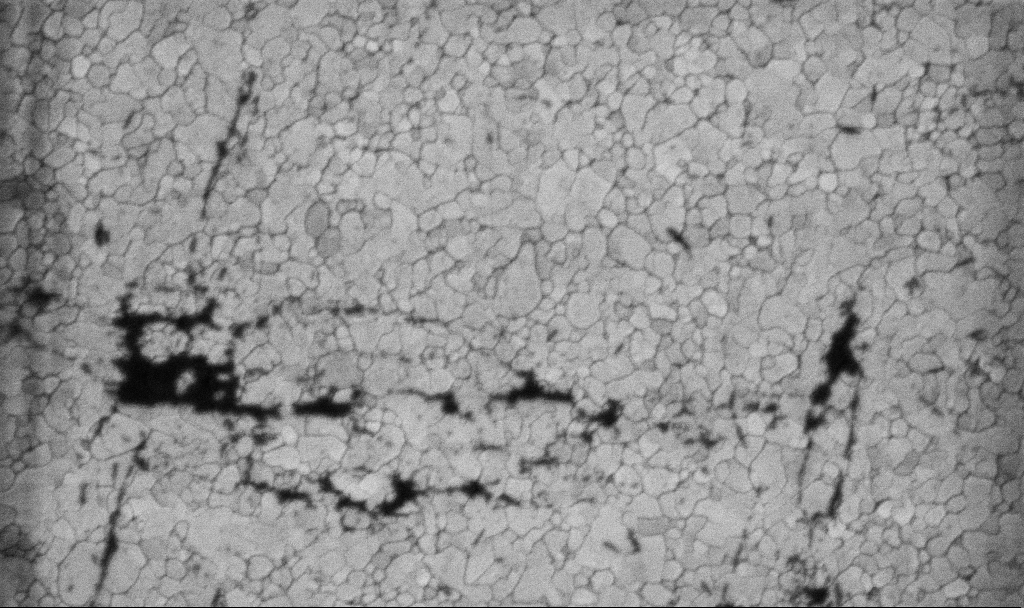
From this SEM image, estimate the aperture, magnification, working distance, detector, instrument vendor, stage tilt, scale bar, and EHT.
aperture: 30 µm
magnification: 100.15 K X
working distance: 3.1 mm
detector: InLens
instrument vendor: Zeiss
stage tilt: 0°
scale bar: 200 nm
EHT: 5 kV